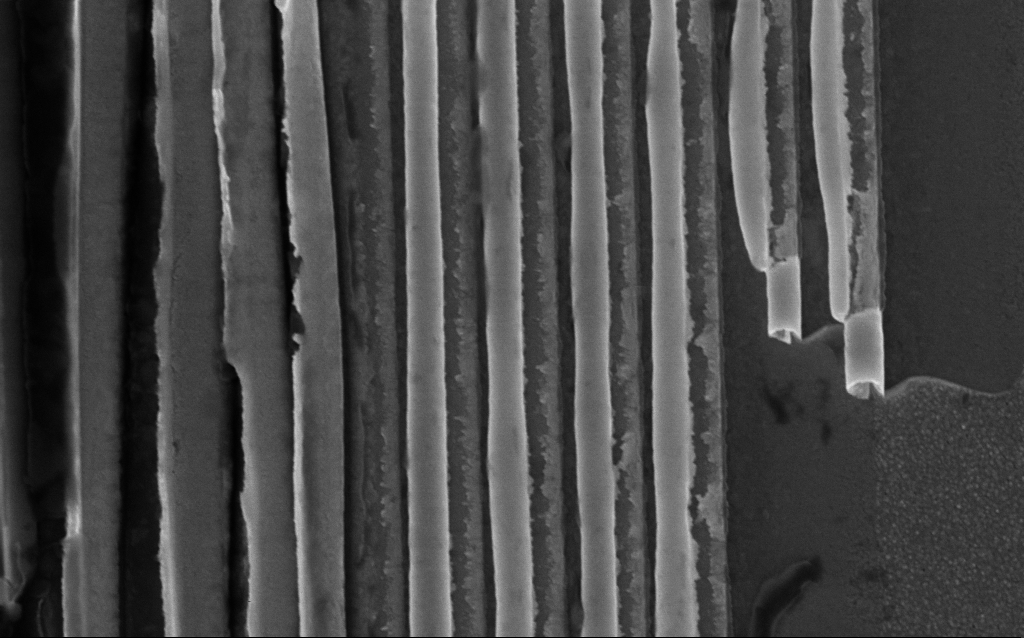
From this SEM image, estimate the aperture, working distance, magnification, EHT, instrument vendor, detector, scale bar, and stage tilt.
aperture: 30 µm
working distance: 8 mm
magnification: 59.97 K X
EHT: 10 kV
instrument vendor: Zeiss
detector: InLens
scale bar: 1000 nm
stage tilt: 0°